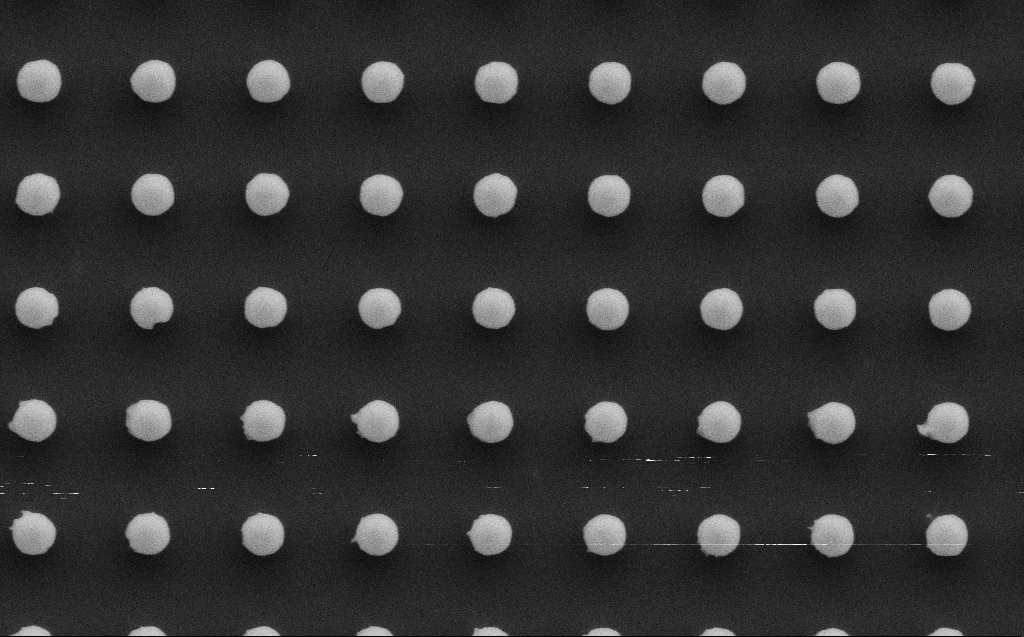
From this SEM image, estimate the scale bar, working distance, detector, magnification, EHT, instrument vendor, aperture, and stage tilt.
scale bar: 1000 nm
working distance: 6 mm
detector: SE2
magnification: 40 K X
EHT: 5 kV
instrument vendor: Zeiss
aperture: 30 µm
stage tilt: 0°